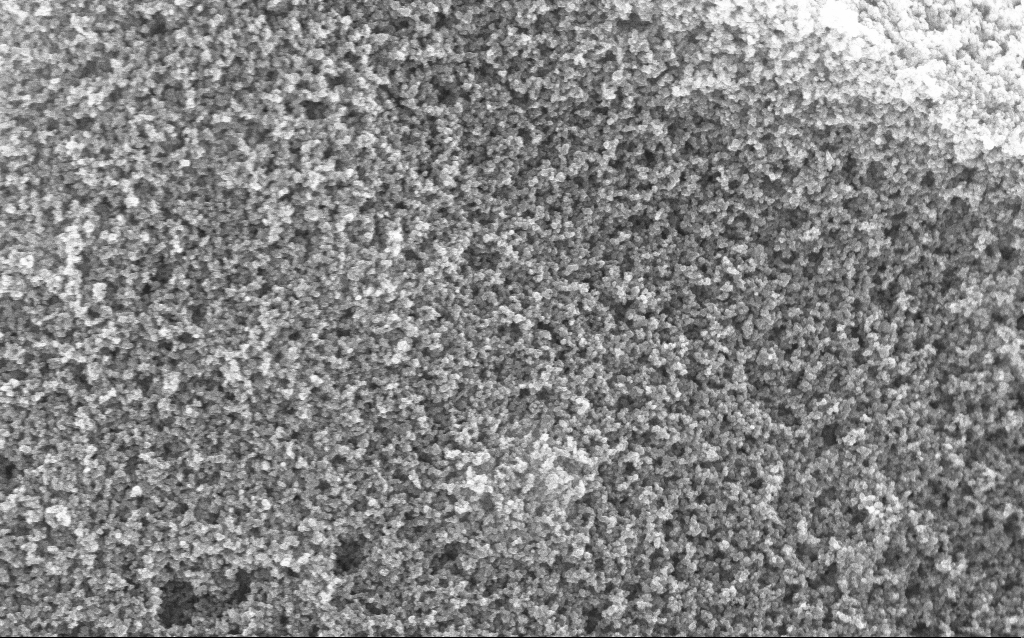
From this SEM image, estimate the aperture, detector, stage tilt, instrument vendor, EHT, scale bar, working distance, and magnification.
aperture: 30 µm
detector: InLens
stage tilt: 0°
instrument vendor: Zeiss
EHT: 10 kV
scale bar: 1000 nm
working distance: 2.7 mm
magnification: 65.04 K X